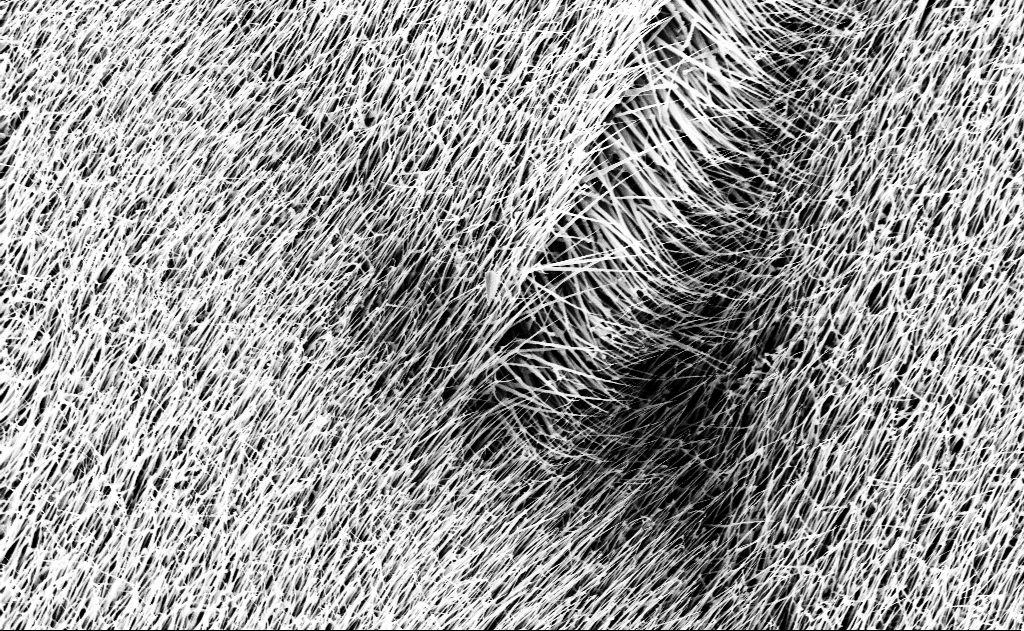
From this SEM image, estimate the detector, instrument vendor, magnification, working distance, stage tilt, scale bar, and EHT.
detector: InLens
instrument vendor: Zeiss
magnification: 10 K X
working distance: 13 mm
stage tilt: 0°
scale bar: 2000 nm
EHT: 10 kV